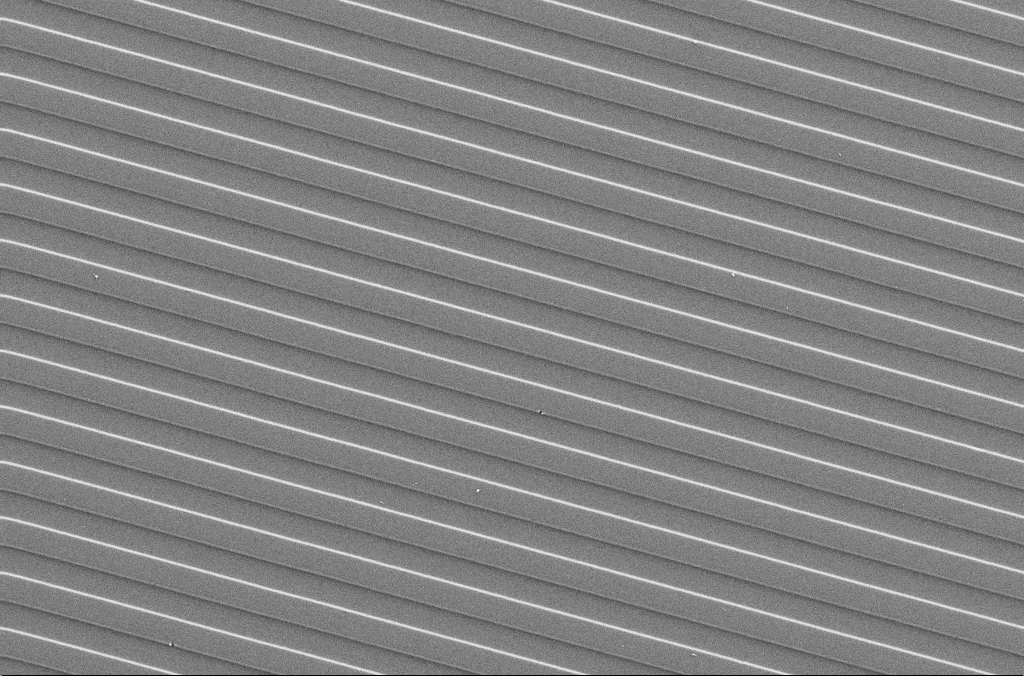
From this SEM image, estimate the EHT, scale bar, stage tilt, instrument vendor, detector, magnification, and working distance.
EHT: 5 kV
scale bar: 20000 nm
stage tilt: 0°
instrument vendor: Zeiss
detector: SE2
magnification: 1 K X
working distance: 4.1 mm